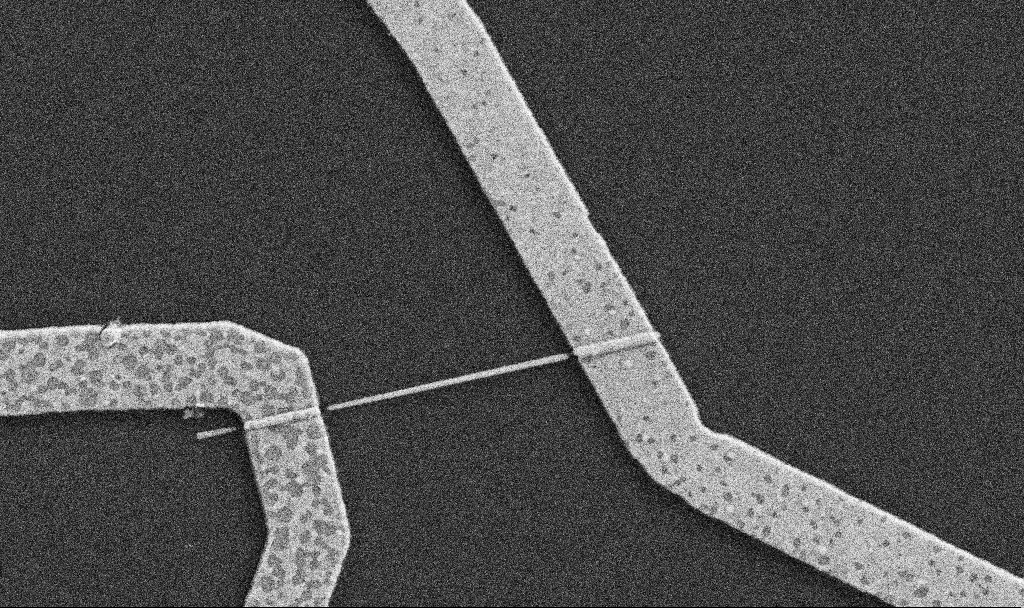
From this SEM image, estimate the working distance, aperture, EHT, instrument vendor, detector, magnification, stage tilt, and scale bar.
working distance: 8.7 mm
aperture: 30 µm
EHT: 5 kV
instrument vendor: Zeiss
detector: SE2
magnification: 30 K X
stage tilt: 0°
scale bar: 1000 nm